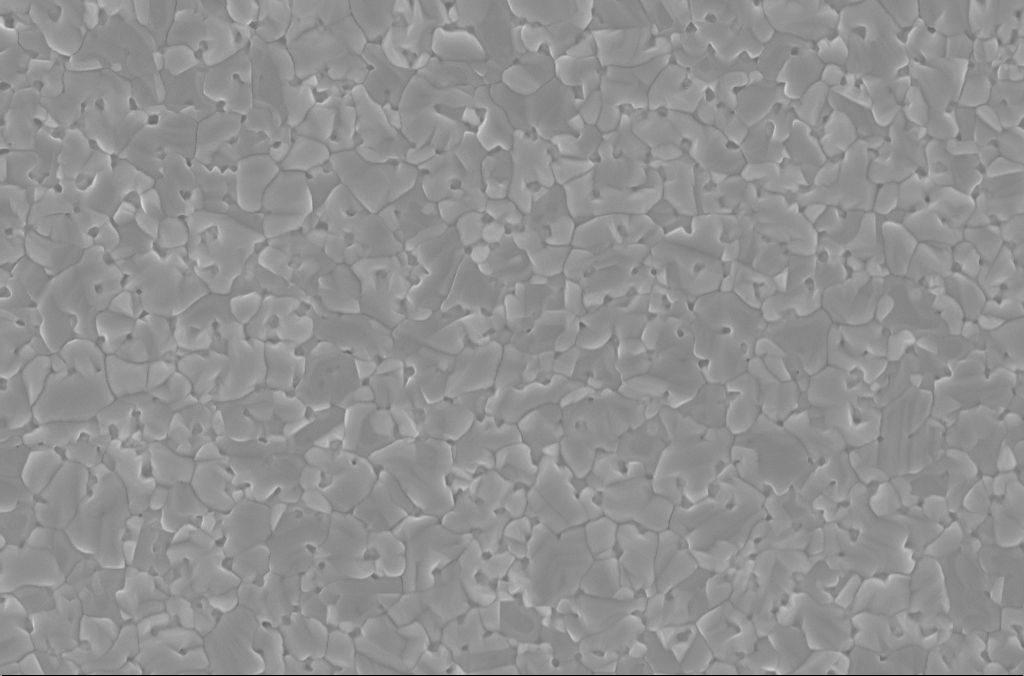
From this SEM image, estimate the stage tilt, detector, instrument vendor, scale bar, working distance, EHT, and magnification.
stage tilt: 0°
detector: InLens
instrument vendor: Zeiss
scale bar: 2000 nm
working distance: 3 mm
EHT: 5 kV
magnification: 30 K X